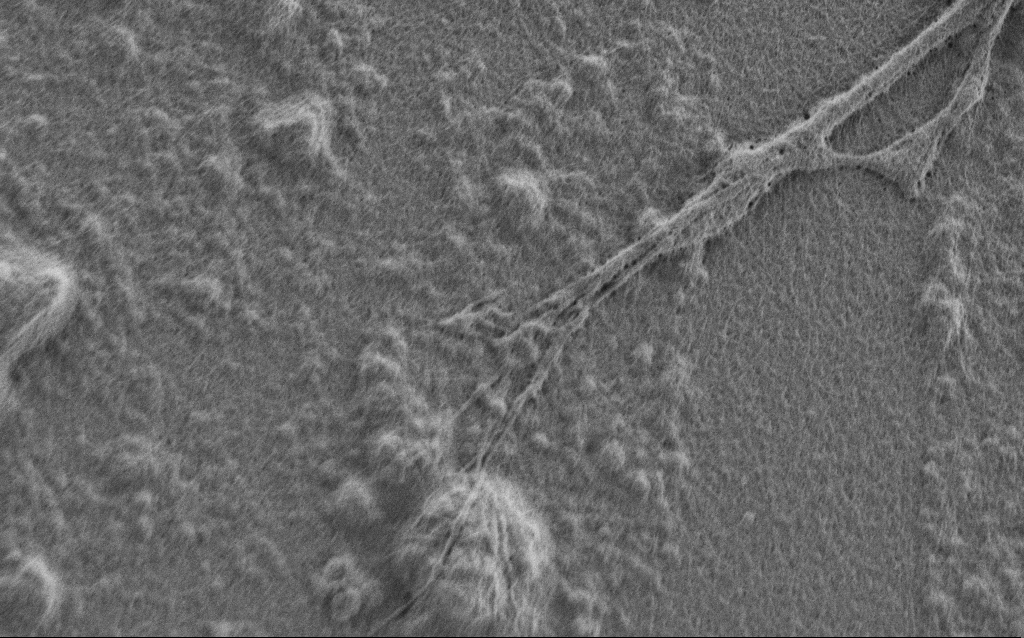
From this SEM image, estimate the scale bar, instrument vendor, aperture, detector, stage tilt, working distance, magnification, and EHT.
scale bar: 2000 nm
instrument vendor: Zeiss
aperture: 30 µm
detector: SE2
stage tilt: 0°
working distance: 7 mm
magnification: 10 K X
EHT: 0.9 kV